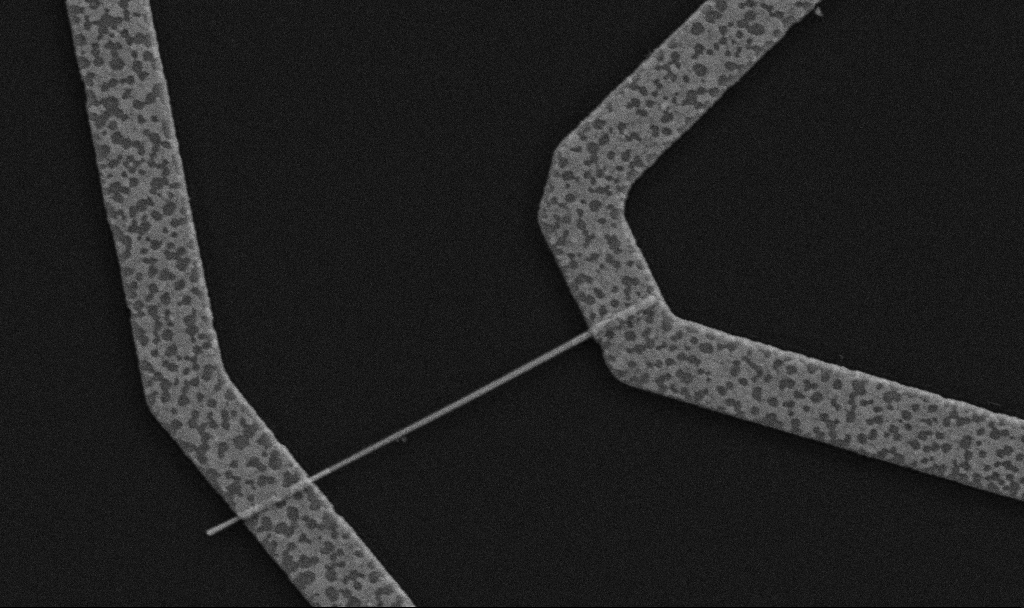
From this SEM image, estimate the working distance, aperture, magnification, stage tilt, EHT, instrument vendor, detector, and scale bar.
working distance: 10.7 mm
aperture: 30 µm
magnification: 30 K X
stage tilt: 0°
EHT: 5 kV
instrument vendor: Zeiss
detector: SE2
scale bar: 1000 nm